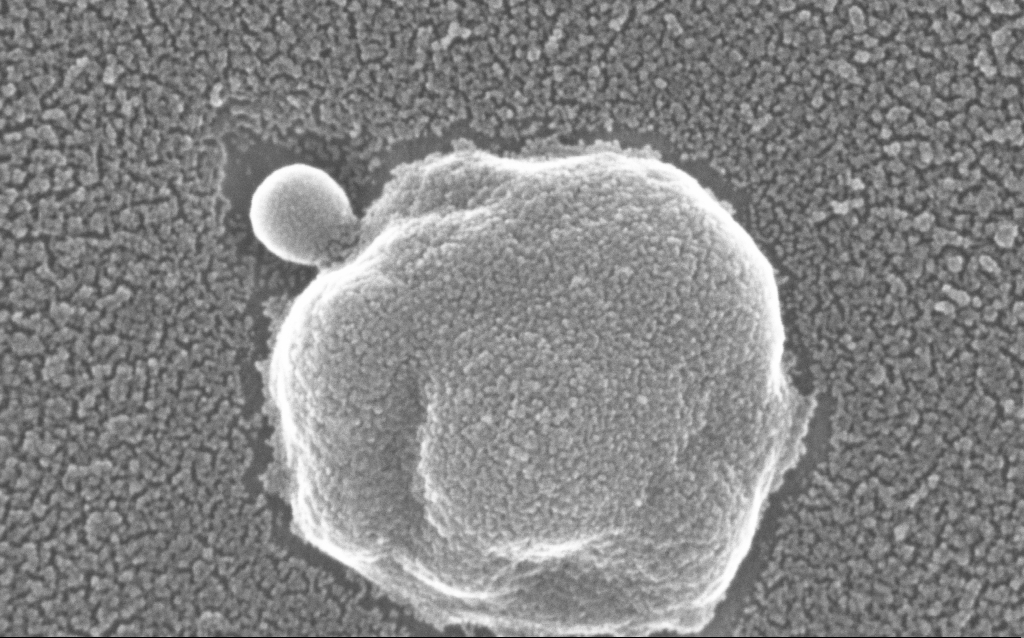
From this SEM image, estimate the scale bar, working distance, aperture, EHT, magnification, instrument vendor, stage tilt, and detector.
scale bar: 100 nm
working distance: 1.5 mm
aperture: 30 µm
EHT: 20 kV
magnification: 500 K X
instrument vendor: Zeiss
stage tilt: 0°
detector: InLens